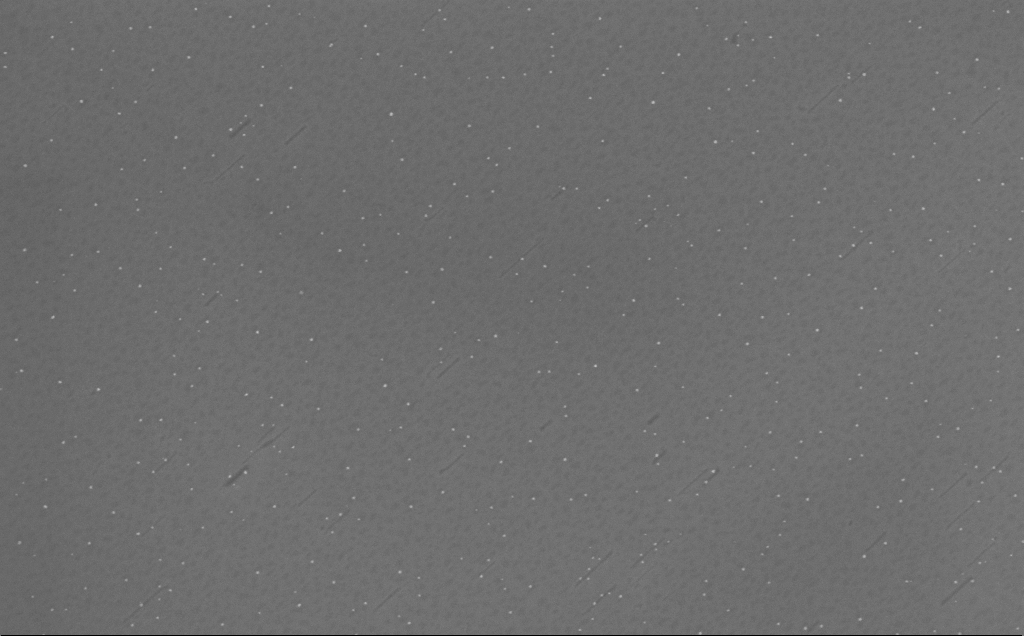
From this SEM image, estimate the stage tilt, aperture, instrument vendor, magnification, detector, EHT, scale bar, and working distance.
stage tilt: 0°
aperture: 30 µm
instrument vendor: Zeiss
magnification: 123.57 K X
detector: InLens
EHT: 10 kV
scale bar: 100 nm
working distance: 6 mm